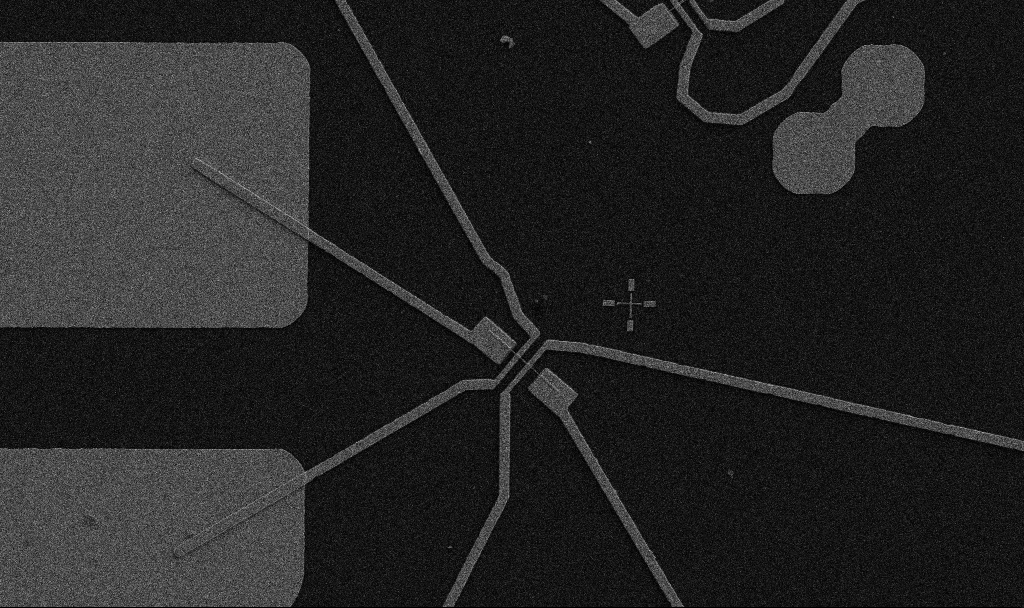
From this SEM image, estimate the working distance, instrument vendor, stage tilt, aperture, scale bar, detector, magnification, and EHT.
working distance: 10.7 mm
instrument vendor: Zeiss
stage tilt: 0°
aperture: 30 µm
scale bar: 10000 nm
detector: SE2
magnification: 5 K X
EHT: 5 kV